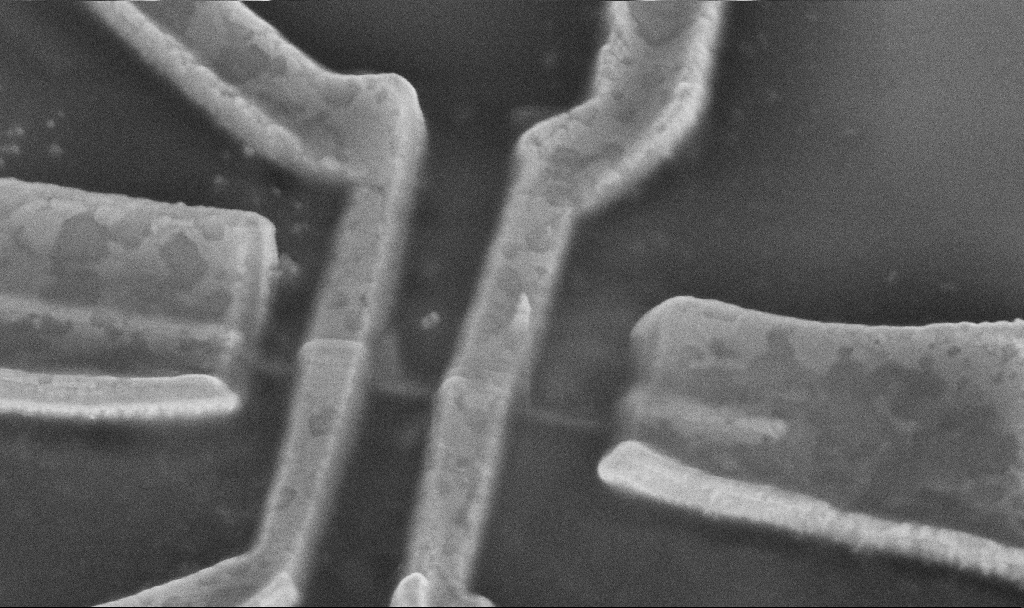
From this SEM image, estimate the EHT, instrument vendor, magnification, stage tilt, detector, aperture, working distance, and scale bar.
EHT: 5 kV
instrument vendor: Zeiss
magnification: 50 K X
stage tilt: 45°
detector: InLens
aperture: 30 µm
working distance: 12.4 mm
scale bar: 1000 nm